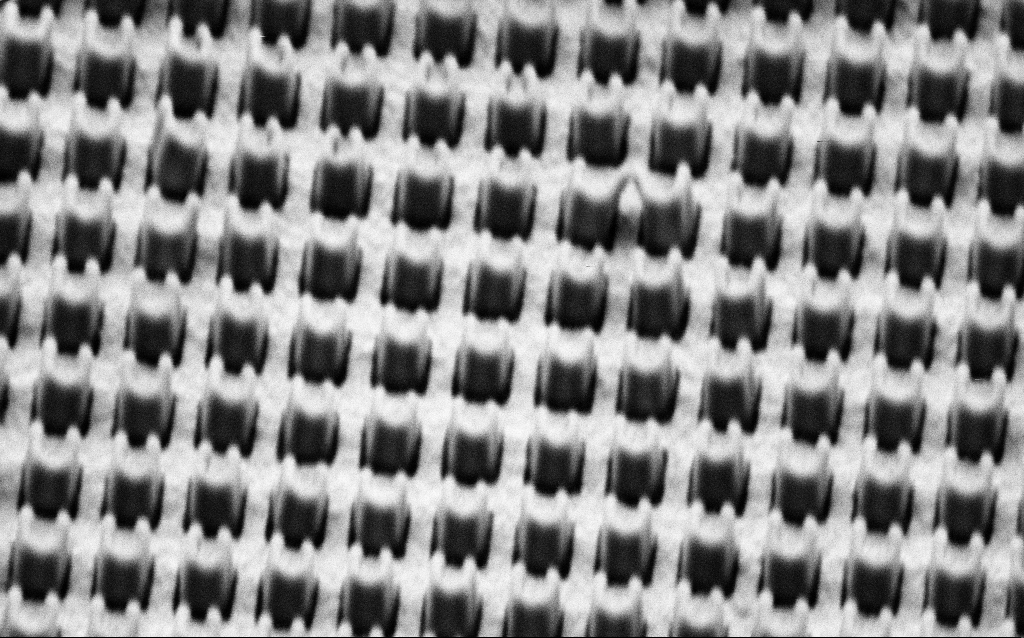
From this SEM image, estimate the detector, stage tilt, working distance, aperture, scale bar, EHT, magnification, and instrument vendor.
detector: SE2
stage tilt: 45°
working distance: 7 mm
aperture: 30 µm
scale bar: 1000 nm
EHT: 1.5 kV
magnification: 65.97 K X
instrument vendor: Zeiss